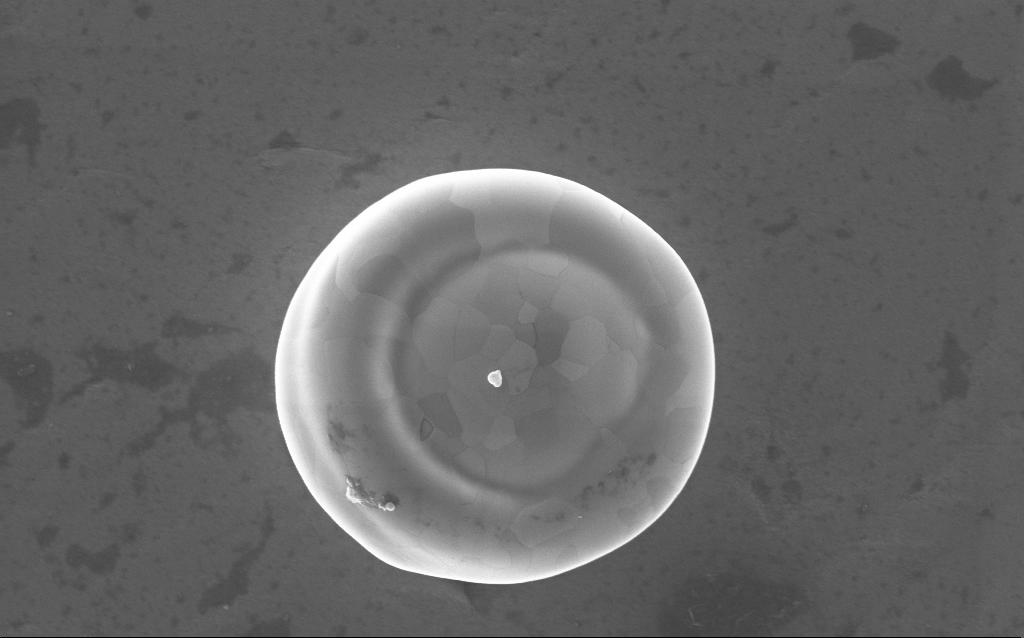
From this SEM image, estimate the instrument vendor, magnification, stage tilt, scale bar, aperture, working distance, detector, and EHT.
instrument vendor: Zeiss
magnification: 36 K X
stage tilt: -0°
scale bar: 2000 nm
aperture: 30 µm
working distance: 2 mm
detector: InLens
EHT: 5 kV